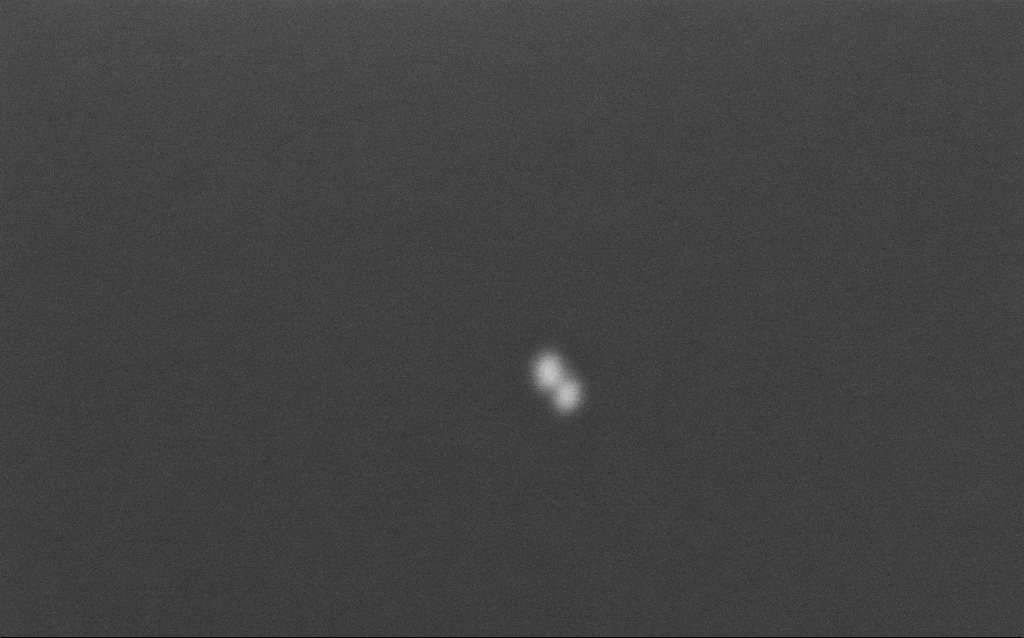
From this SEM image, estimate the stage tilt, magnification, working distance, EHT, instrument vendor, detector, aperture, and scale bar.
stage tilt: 0°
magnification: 682.17 K X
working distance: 2.9 mm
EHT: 4 kV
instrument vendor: Zeiss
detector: InLens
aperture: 30 µm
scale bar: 100 nm